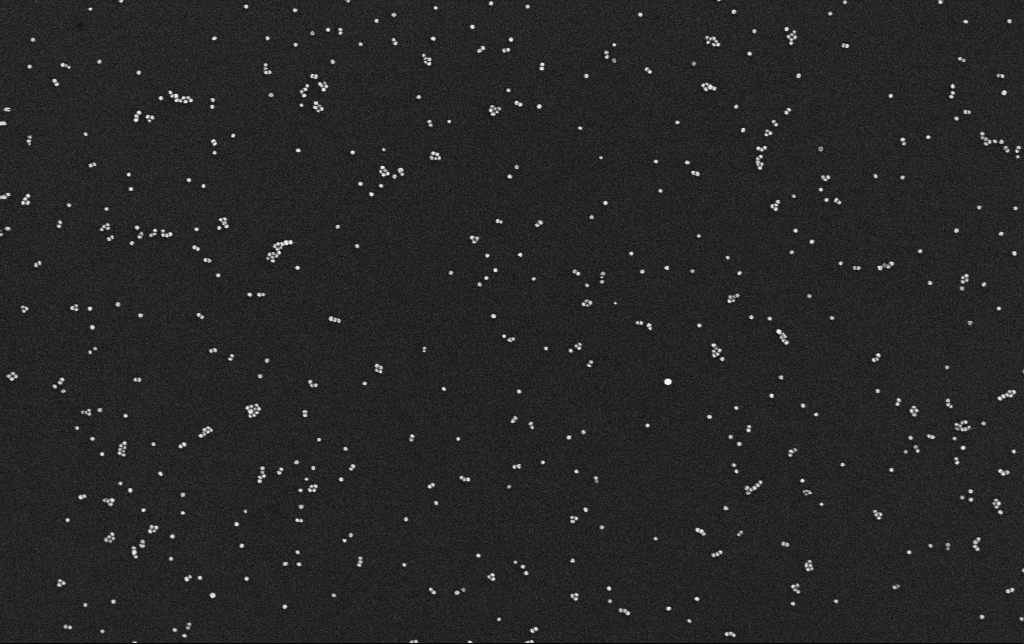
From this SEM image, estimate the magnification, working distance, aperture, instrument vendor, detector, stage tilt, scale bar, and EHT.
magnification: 100 K X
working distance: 3.1 mm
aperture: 30 µm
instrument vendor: Zeiss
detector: InLens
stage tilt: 0°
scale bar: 200 nm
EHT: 10 kV